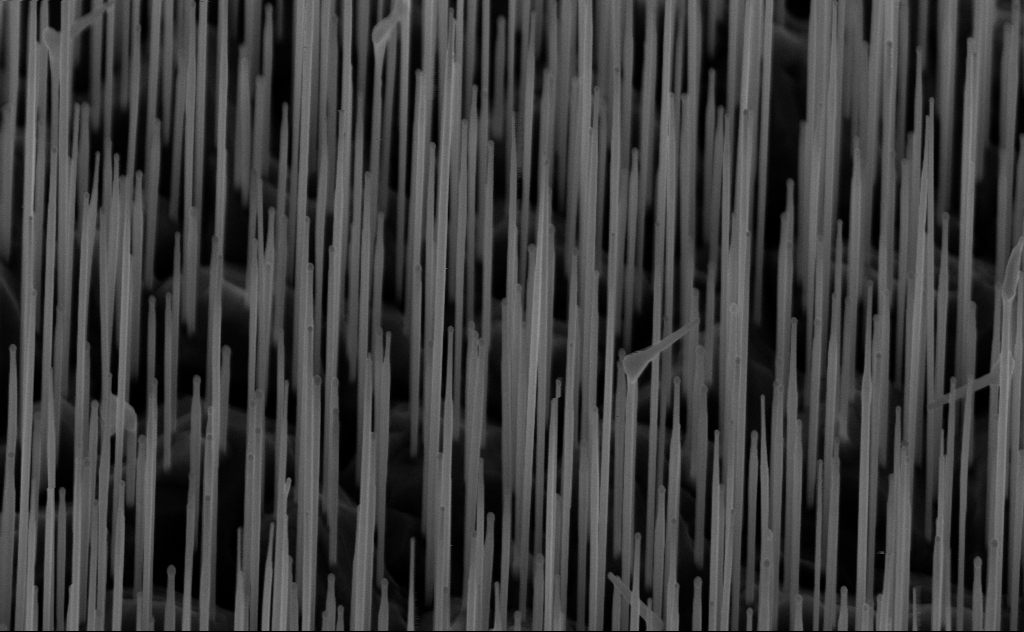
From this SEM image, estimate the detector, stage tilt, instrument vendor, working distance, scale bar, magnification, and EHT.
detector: InLens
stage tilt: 45°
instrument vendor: Zeiss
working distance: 6 mm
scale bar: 1000 nm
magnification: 40 K X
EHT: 10 kV